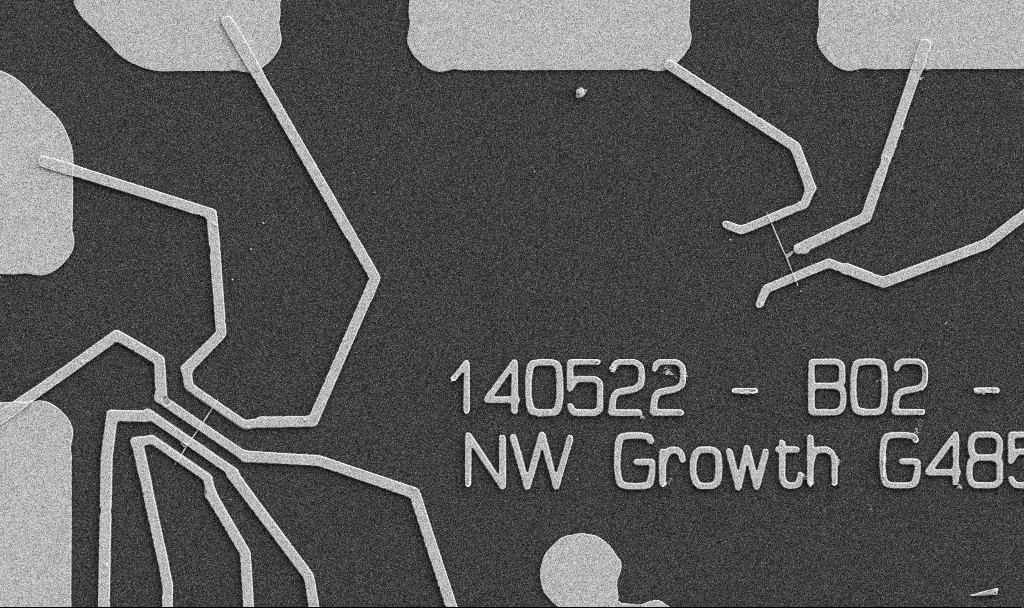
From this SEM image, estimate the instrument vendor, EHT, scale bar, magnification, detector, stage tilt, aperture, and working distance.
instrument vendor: Zeiss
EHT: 5 kV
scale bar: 10000 nm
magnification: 5 K X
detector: SE2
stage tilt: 0°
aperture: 30 µm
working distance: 10.7 mm